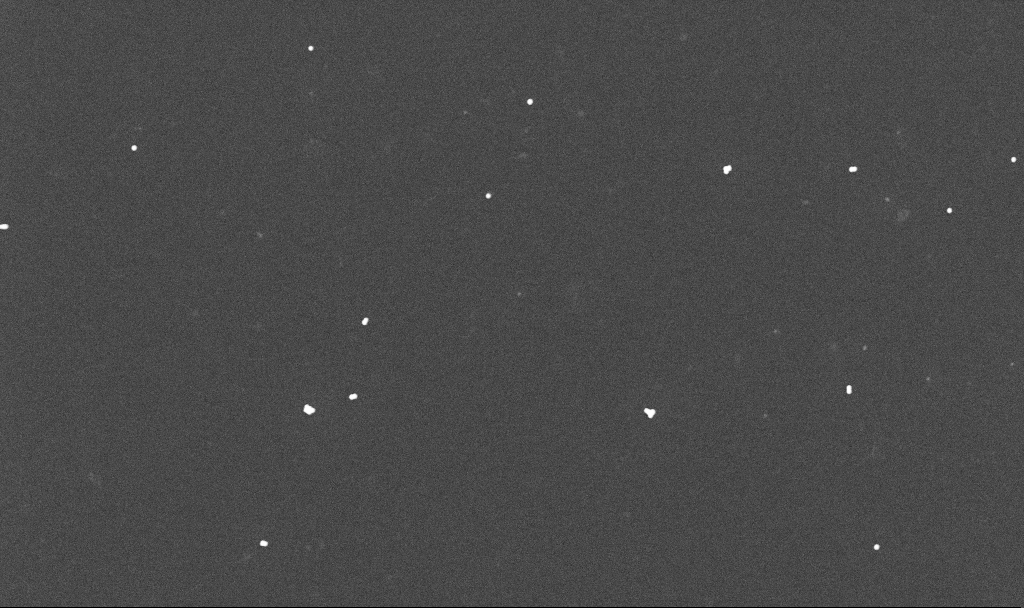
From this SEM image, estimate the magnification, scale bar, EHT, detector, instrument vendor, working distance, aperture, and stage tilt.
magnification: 20 K X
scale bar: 1000 nm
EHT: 10 kV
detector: InLens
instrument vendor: Zeiss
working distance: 3.4 mm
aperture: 30 µm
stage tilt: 0°